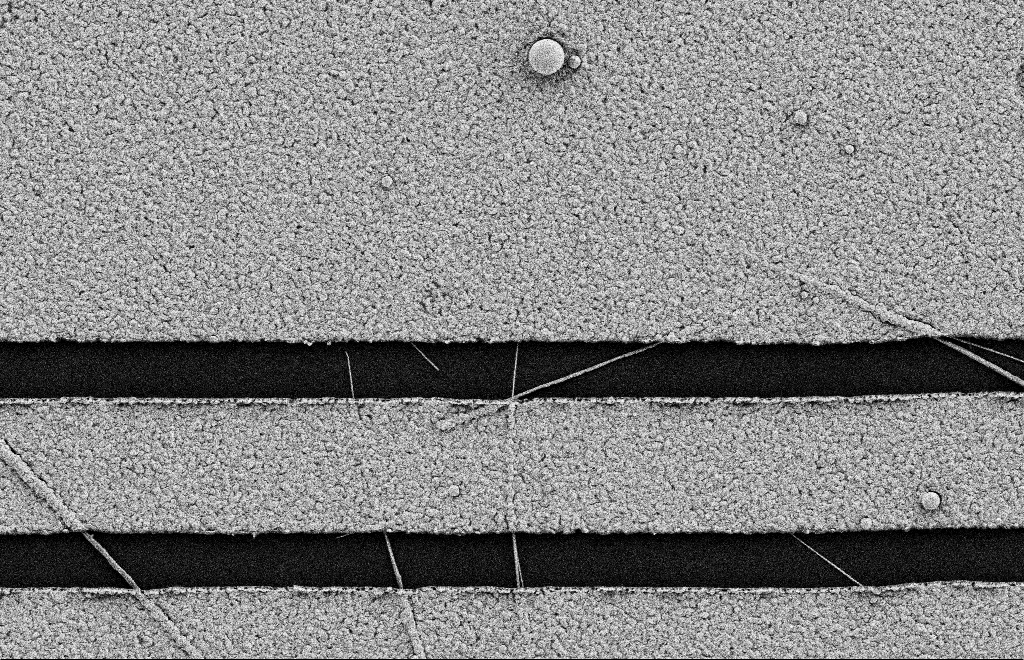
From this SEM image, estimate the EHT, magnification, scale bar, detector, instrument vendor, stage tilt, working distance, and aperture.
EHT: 2 kV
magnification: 17.33 K X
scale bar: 2000 nm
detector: SE2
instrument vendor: Zeiss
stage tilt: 0°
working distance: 11 mm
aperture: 20 µm